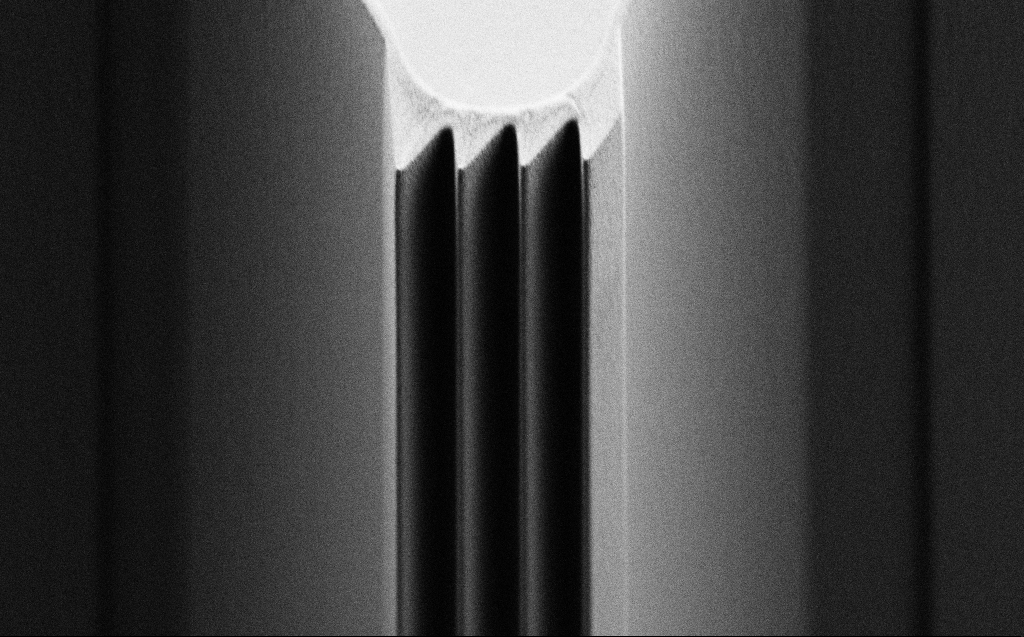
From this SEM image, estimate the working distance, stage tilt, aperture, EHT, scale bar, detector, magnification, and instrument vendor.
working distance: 4 mm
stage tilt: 45°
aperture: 30 µm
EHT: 0.9 kV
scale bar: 10000 nm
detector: SE2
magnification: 2.05 K X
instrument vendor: Zeiss